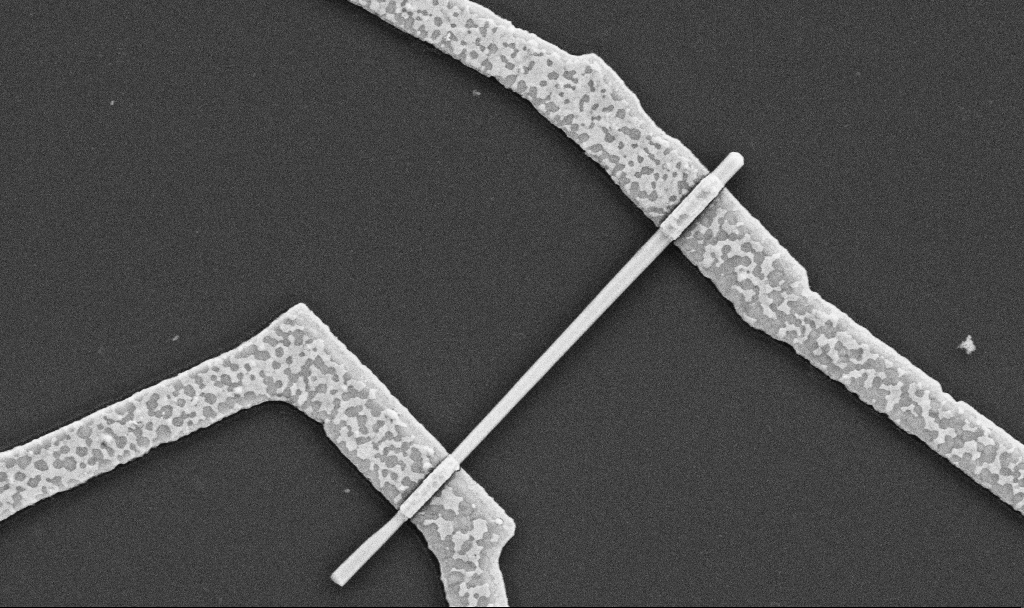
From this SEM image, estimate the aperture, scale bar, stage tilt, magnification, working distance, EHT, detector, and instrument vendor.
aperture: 30 µm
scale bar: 1000 nm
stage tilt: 0°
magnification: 30 K X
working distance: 8.7 mm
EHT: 5 kV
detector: SE2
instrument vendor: Zeiss